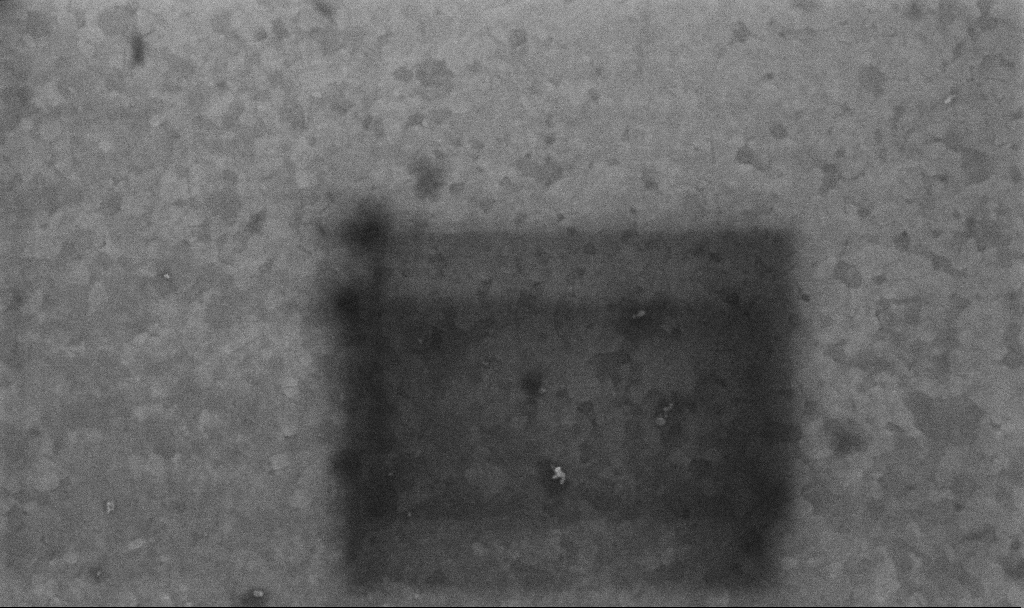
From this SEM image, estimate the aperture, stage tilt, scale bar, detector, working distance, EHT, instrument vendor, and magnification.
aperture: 30 µm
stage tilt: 0°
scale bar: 200 nm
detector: InLens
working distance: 3.4 mm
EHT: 10 kV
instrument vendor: Zeiss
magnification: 99.94 K X